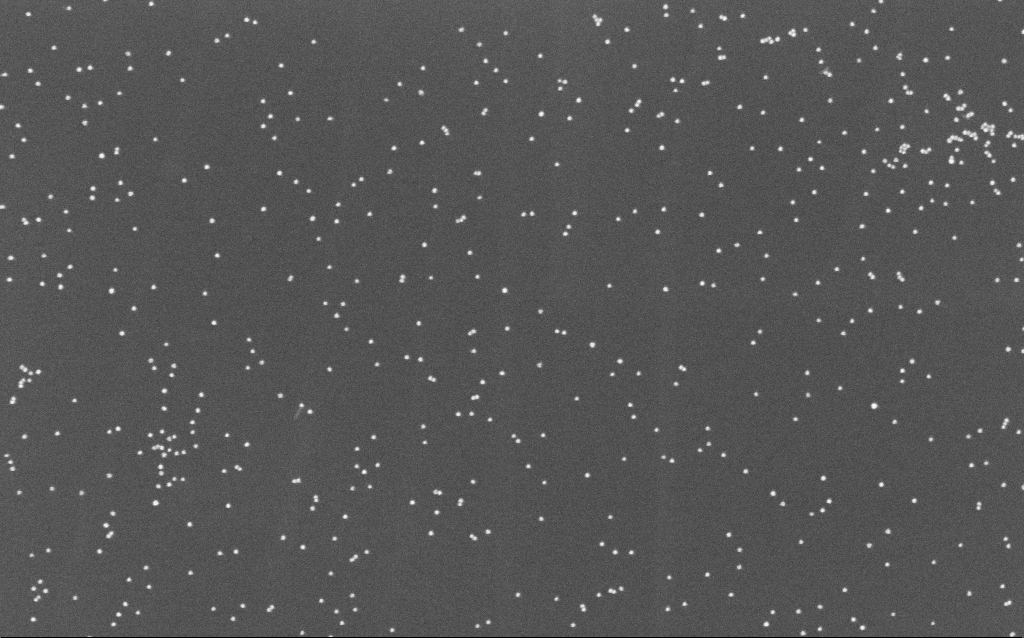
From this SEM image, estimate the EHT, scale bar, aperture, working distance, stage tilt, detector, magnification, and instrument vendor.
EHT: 10 kV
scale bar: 200 nm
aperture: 30 µm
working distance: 6.6 mm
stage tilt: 0°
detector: InLens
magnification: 100 K X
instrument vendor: Zeiss